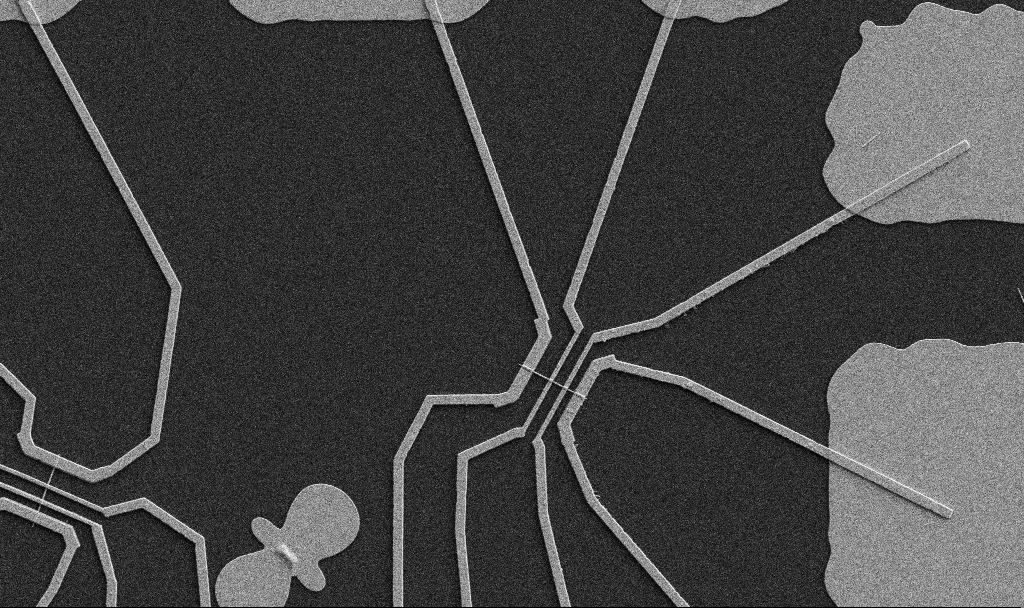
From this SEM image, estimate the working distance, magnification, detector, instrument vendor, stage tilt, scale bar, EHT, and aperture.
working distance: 10.7 mm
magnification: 5 K X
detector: SE2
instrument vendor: Zeiss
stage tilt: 0°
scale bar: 10000 nm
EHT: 5 kV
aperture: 30 µm